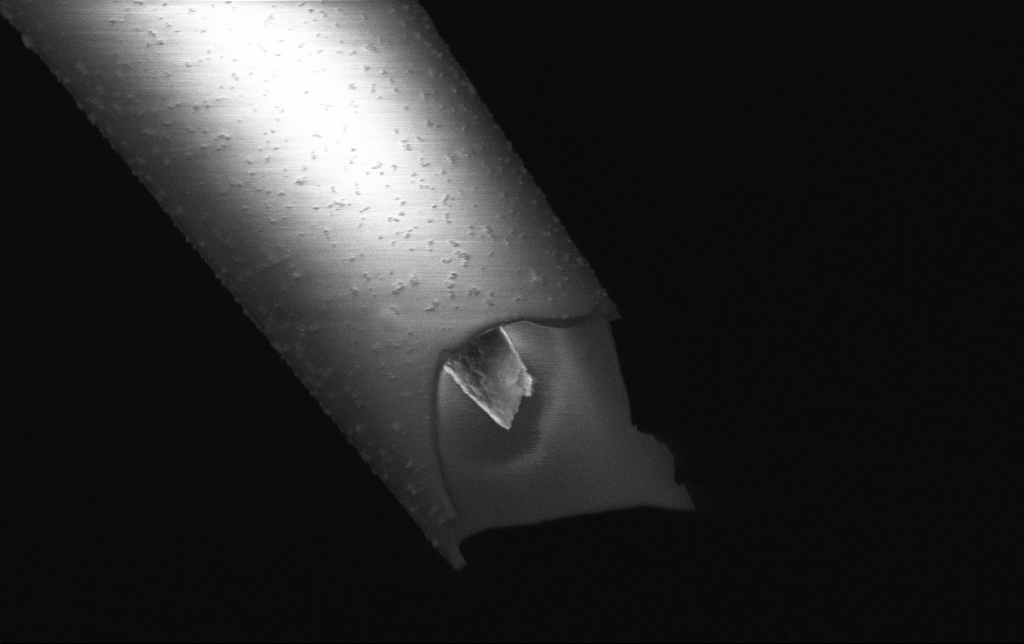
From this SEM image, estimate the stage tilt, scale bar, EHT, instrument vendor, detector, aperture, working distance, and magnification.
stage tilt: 0°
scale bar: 2000 nm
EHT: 3 kV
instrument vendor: Zeiss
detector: InLens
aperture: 30 µm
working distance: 6.7 mm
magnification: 25 K X